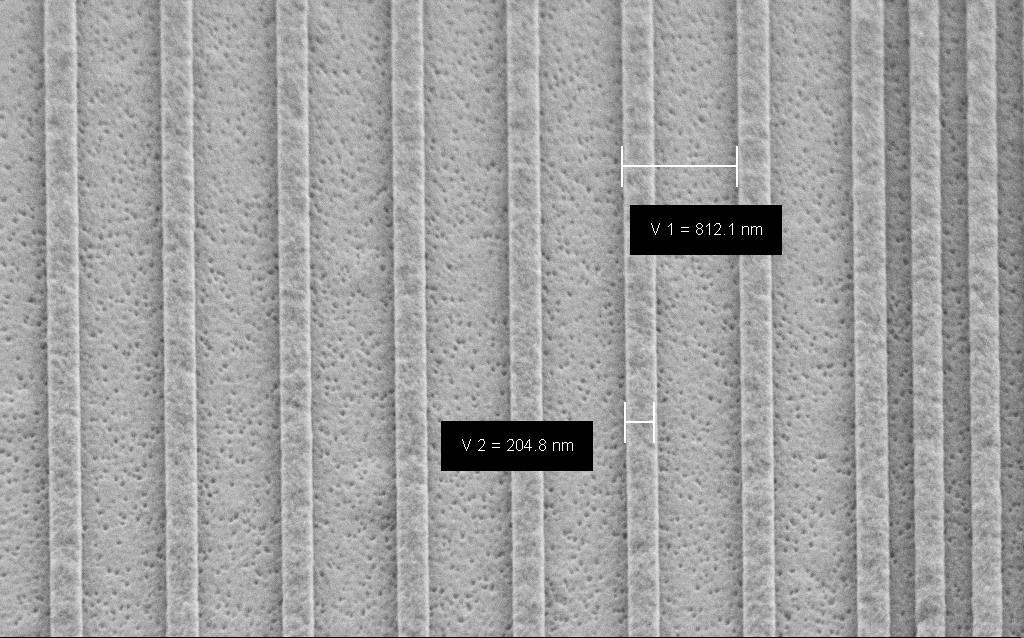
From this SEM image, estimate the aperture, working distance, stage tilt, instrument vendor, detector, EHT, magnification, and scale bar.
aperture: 30 µm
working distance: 6.6 mm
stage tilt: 45°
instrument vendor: Zeiss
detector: SE2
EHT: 3 kV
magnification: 51.99 K X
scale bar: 1000 nm